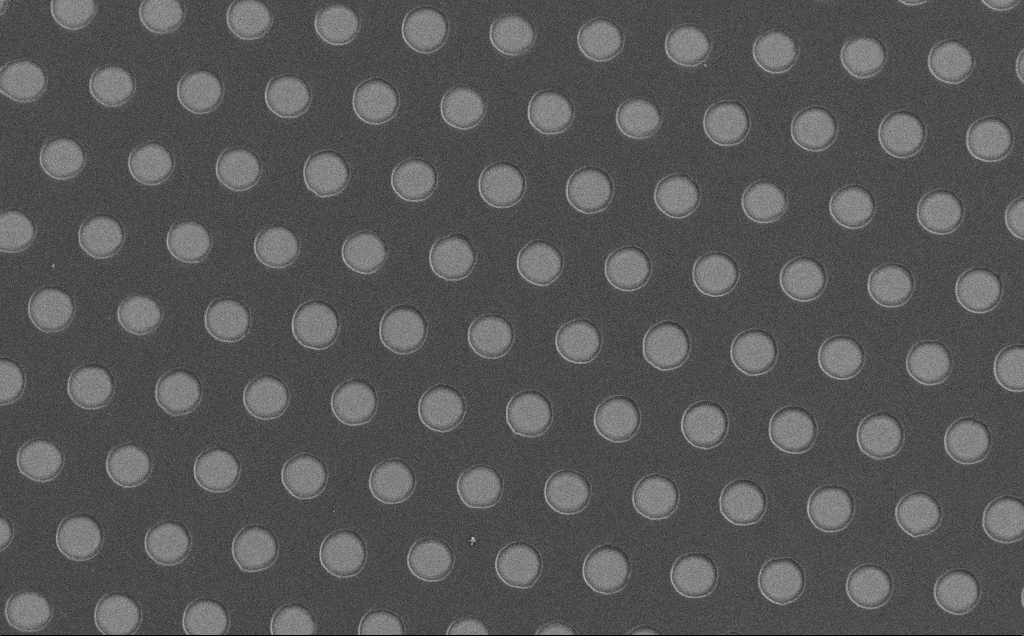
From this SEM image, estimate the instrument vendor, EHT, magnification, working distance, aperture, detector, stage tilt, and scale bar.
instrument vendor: Zeiss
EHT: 5 kV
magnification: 0.651 K X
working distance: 6 mm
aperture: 30 µm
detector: SE2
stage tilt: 0°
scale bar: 100000 nm